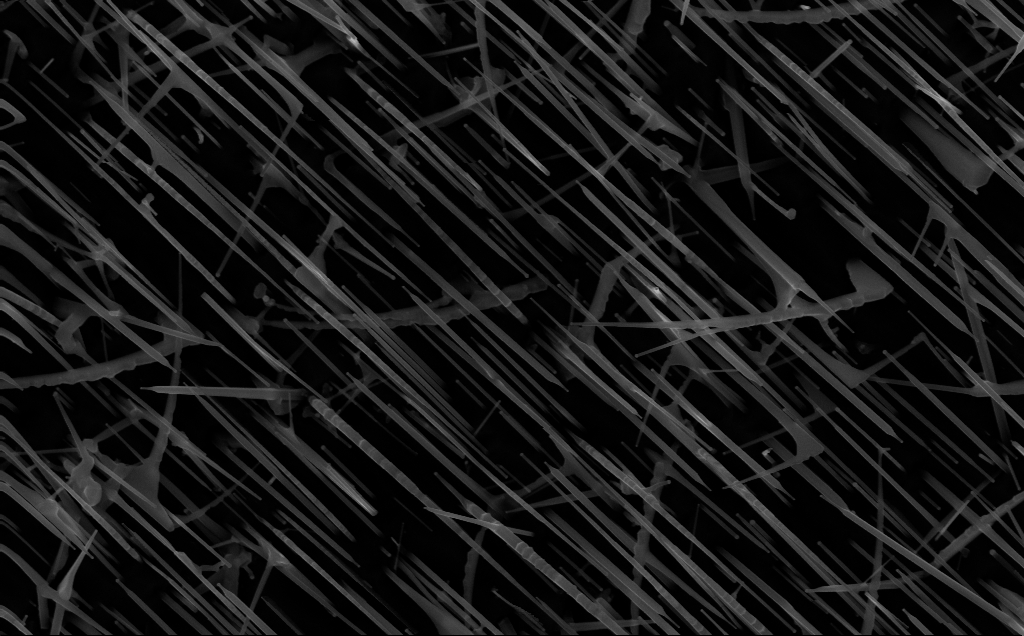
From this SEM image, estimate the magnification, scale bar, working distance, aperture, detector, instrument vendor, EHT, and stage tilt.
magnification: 40 K X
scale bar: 1000 nm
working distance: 5 mm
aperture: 30 µm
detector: InLens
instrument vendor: Zeiss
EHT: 10 kV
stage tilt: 0°